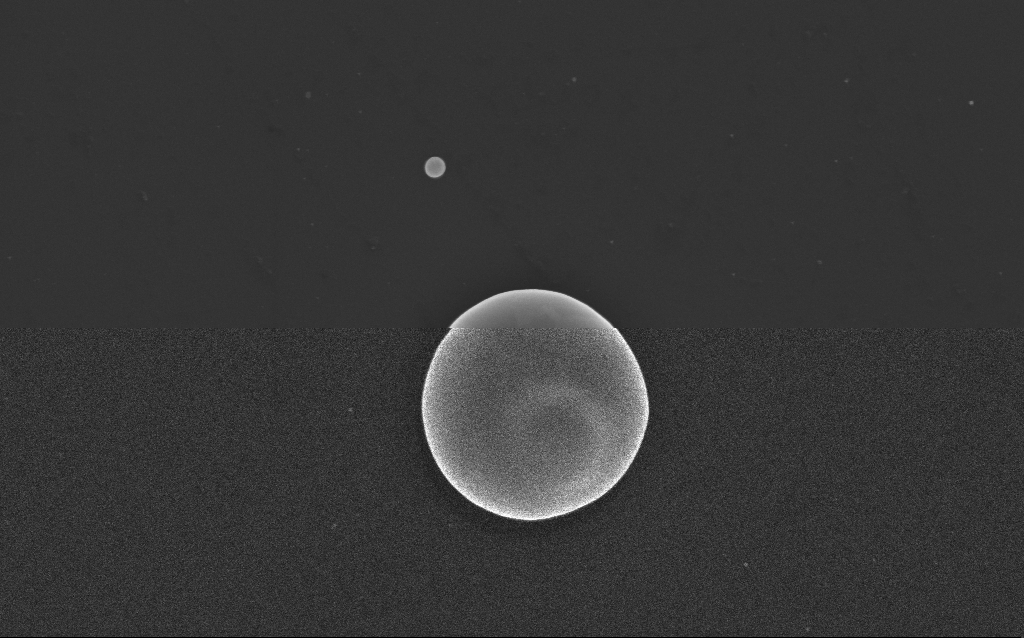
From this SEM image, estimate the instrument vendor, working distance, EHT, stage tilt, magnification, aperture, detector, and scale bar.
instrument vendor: Zeiss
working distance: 3 mm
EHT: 10 kV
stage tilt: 0°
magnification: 35.25 K X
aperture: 30 µm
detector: InLens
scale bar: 2000 nm